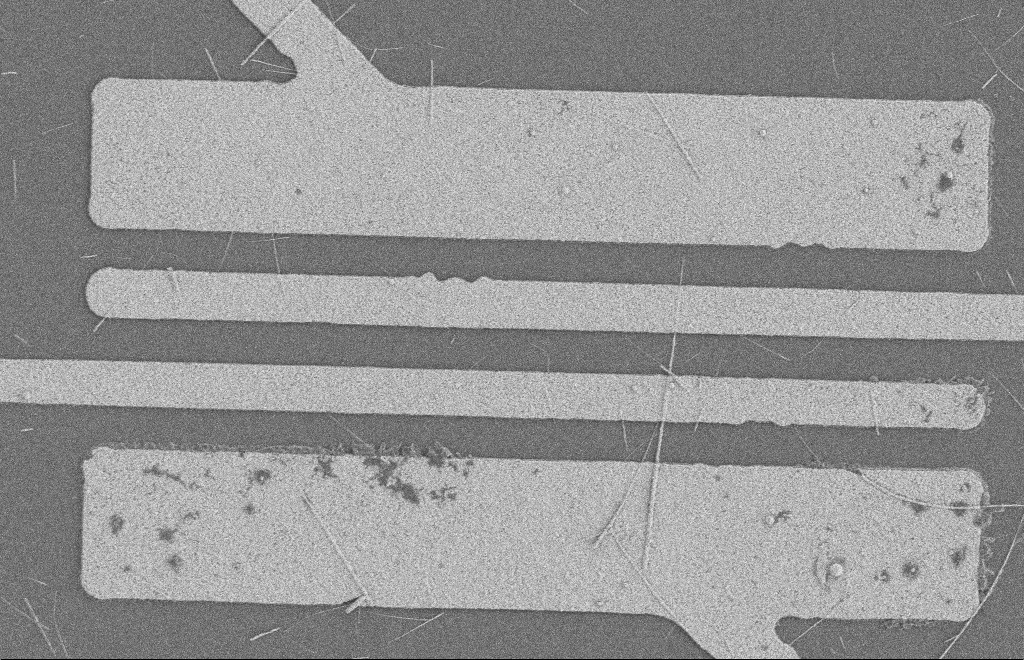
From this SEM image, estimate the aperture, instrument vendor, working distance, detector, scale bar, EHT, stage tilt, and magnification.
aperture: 20 µm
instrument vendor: Zeiss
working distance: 8 mm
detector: SE2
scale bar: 2000 nm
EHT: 2 kV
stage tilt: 0°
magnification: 5.44 K X